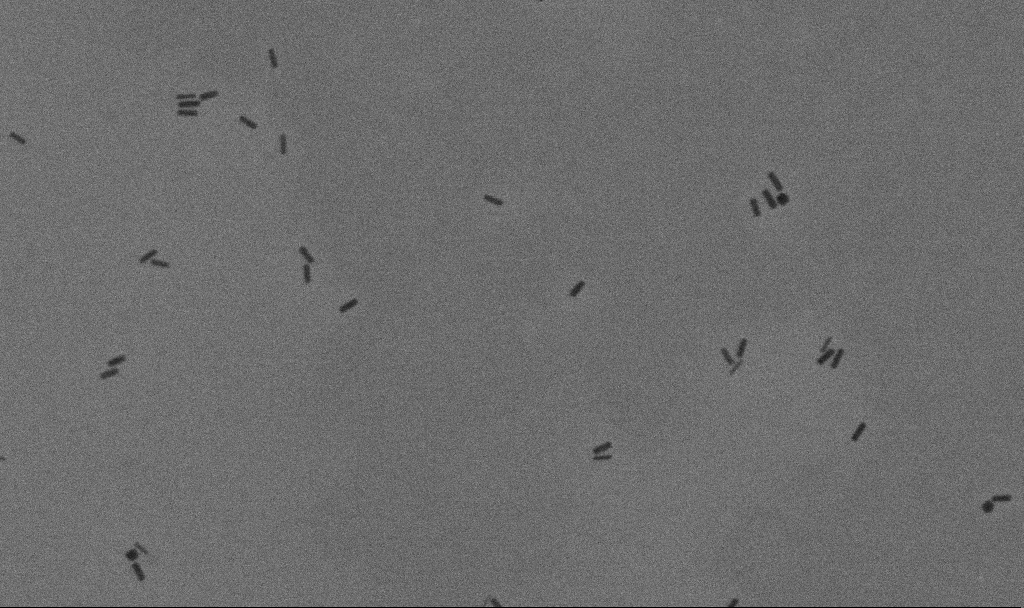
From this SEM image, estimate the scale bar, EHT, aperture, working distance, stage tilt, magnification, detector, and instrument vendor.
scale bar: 200 nm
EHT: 10 kV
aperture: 30 µm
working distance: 11.3 mm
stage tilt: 0°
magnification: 100 K X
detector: SE2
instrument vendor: Zeiss